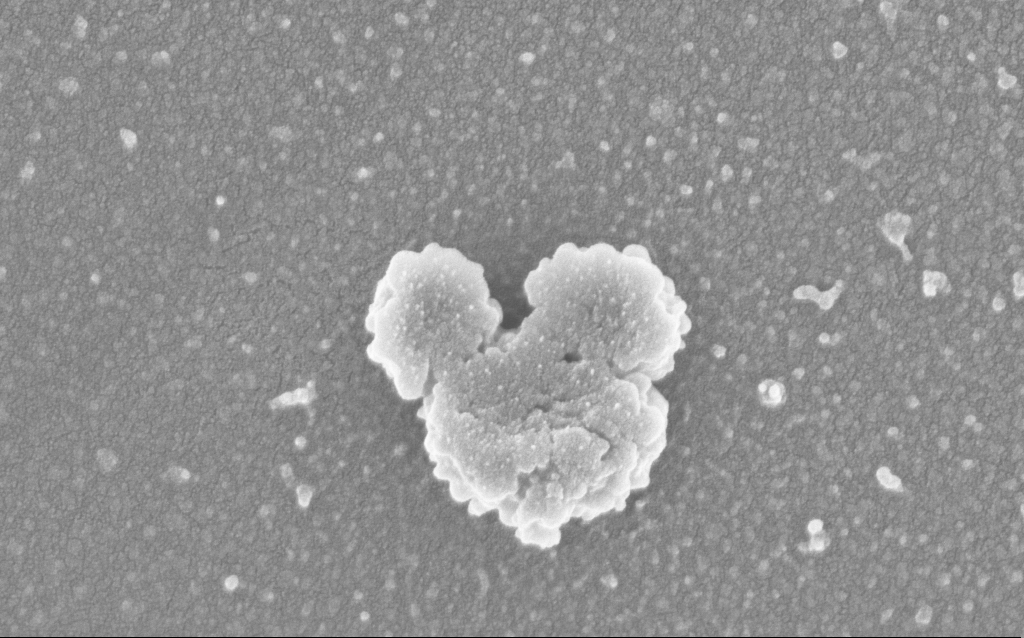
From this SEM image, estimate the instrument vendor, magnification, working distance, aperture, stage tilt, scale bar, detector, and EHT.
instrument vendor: Zeiss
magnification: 150 K X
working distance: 2.5 mm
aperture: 30 µm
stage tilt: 0°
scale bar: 200 nm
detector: InLens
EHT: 3 kV